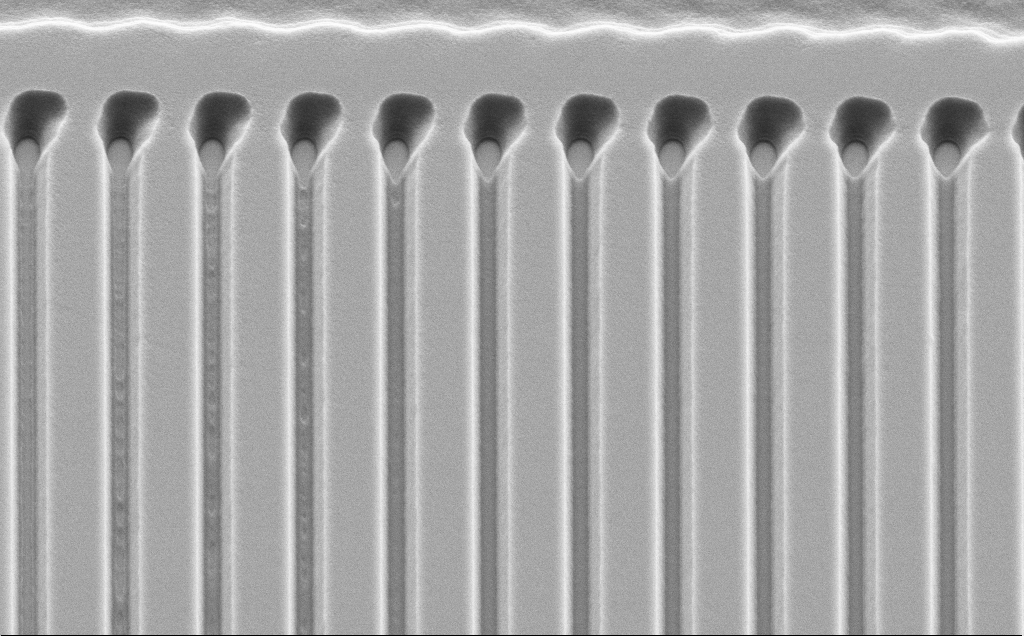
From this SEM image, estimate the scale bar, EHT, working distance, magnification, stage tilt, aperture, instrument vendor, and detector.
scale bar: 2000 nm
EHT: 5 kV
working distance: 10 mm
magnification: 8.39 K X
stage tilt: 45°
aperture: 30 µm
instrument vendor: Zeiss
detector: SE2